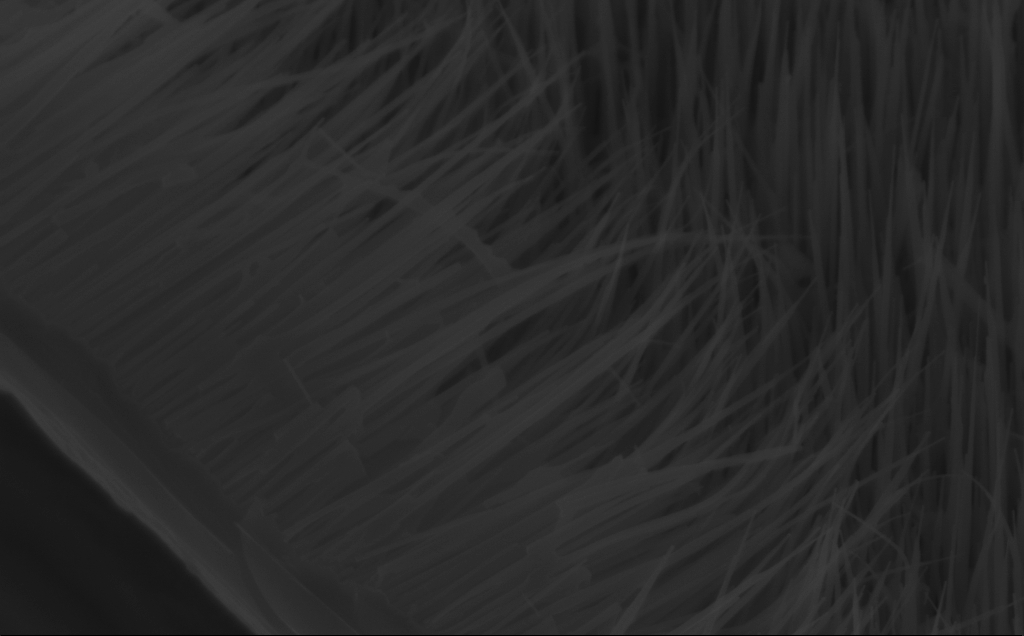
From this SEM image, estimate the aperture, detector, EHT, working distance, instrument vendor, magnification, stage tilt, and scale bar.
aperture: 30 µm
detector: InLens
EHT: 10 kV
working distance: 8 mm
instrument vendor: Zeiss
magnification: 80 K X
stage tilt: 45°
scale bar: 200 nm